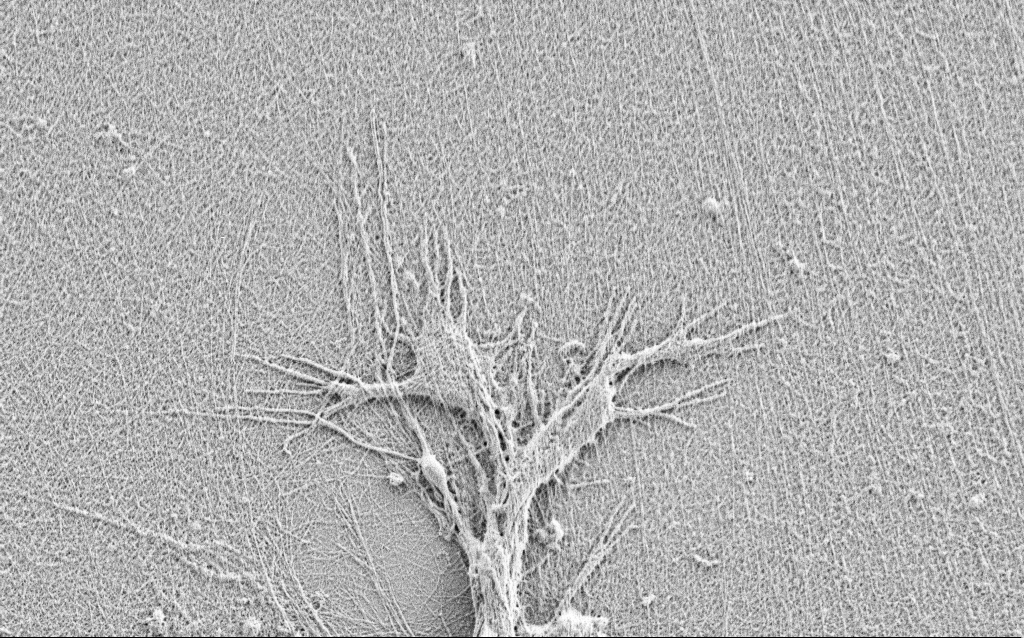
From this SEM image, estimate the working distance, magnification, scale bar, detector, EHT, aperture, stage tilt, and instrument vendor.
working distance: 3 mm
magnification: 10 K X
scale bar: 2000 nm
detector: SE2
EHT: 0.9 kV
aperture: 30 µm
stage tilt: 0°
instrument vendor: Zeiss